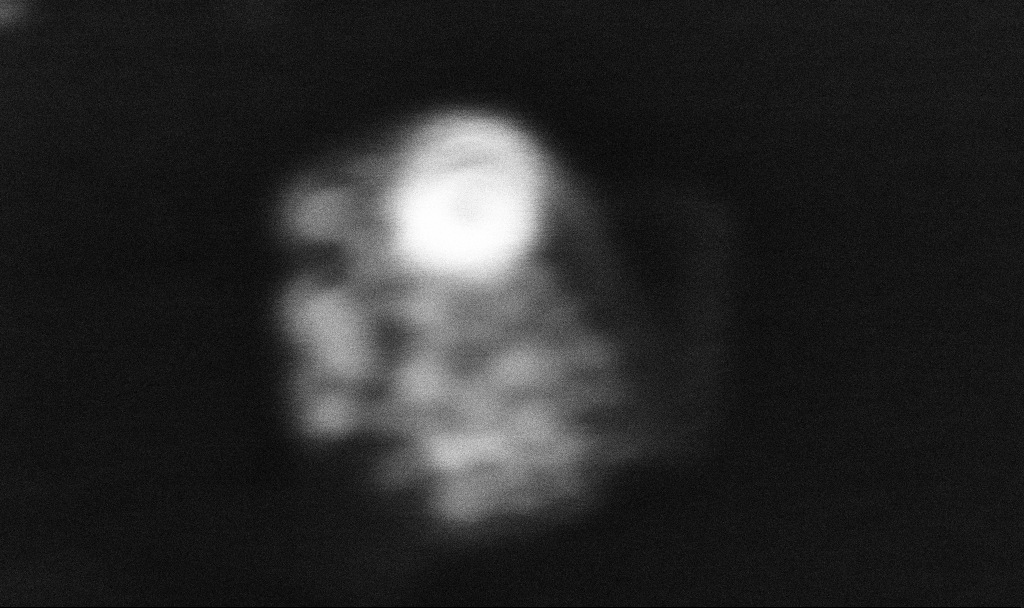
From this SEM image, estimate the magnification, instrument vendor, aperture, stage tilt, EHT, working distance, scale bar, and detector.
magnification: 1112.58 K X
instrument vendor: Zeiss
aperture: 30 µm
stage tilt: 0°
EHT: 10 kV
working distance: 3.4 mm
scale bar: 20 nm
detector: InLens